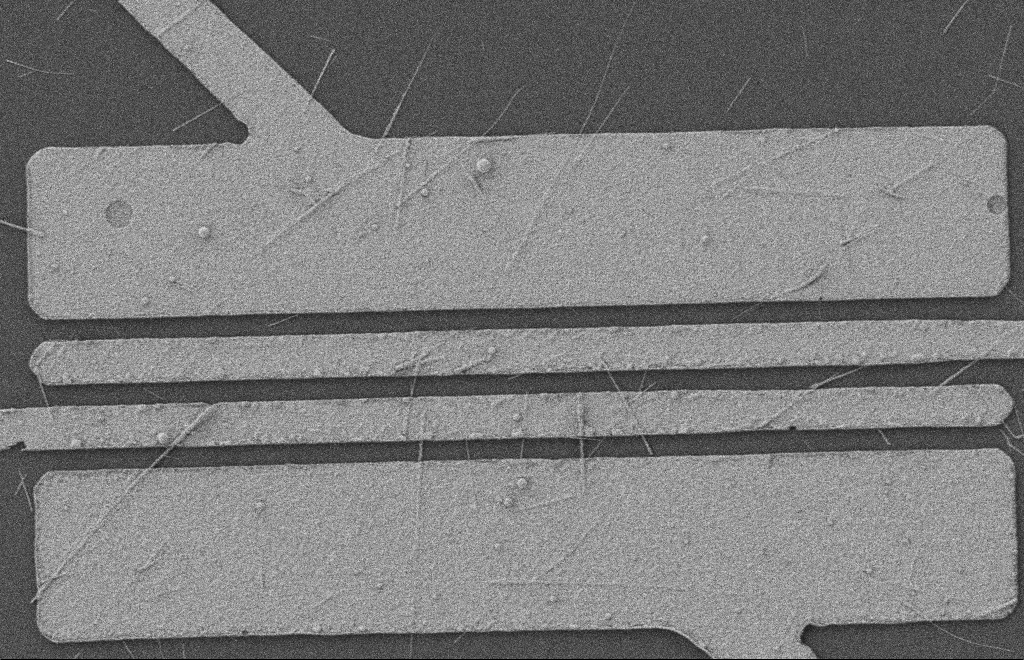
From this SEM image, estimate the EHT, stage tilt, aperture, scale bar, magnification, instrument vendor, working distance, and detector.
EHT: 2 kV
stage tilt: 0°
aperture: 20 µm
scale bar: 2000 nm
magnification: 5.91 K X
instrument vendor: Zeiss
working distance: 8 mm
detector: SE2